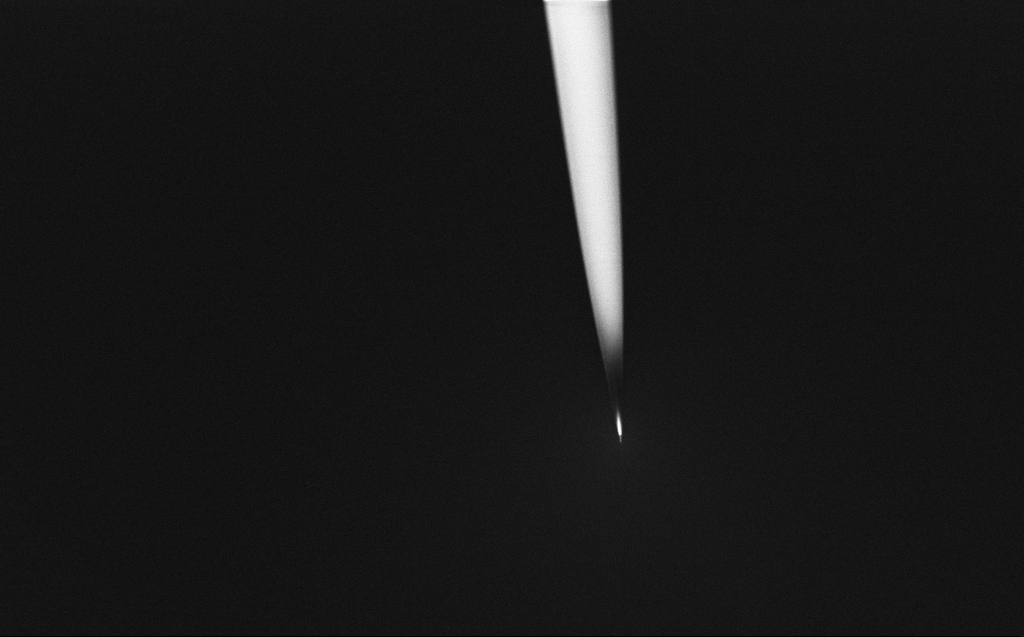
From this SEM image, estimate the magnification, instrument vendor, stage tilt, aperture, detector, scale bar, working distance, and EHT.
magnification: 1 K X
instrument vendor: Zeiss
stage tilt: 45°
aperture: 30 µm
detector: InLens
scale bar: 20000 nm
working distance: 6 mm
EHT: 2 kV